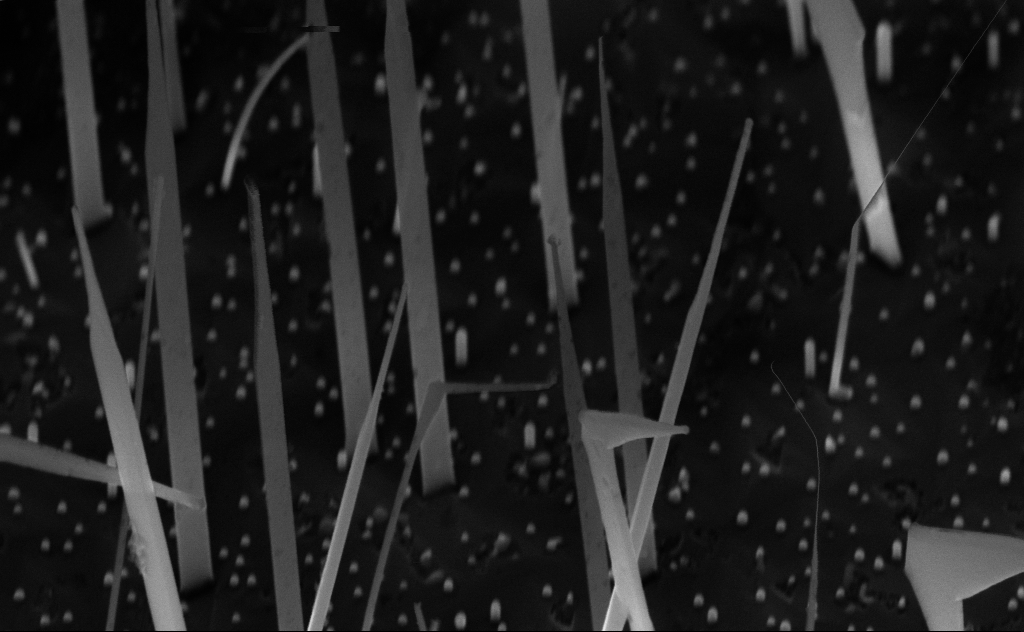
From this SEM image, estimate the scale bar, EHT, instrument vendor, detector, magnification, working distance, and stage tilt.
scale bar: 200 nm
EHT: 10 kV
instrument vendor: Zeiss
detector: InLens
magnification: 80 K X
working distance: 6 mm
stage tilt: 45°